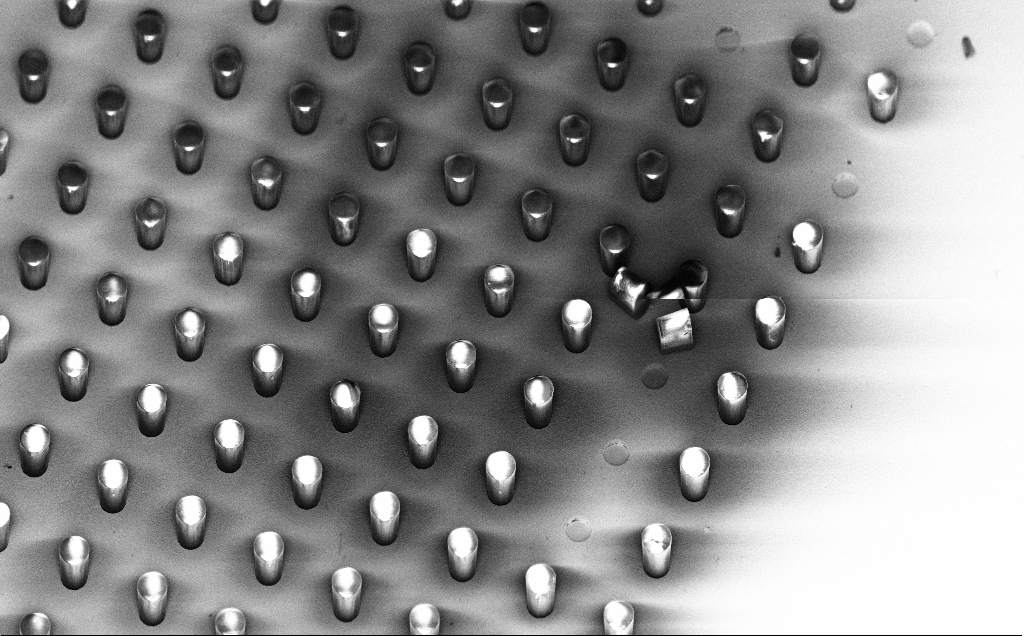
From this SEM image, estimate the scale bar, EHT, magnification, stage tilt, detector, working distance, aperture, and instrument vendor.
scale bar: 100000 nm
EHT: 5 kV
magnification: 0.534 K X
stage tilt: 45°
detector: InLens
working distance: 8 mm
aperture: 30 µm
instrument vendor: Zeiss